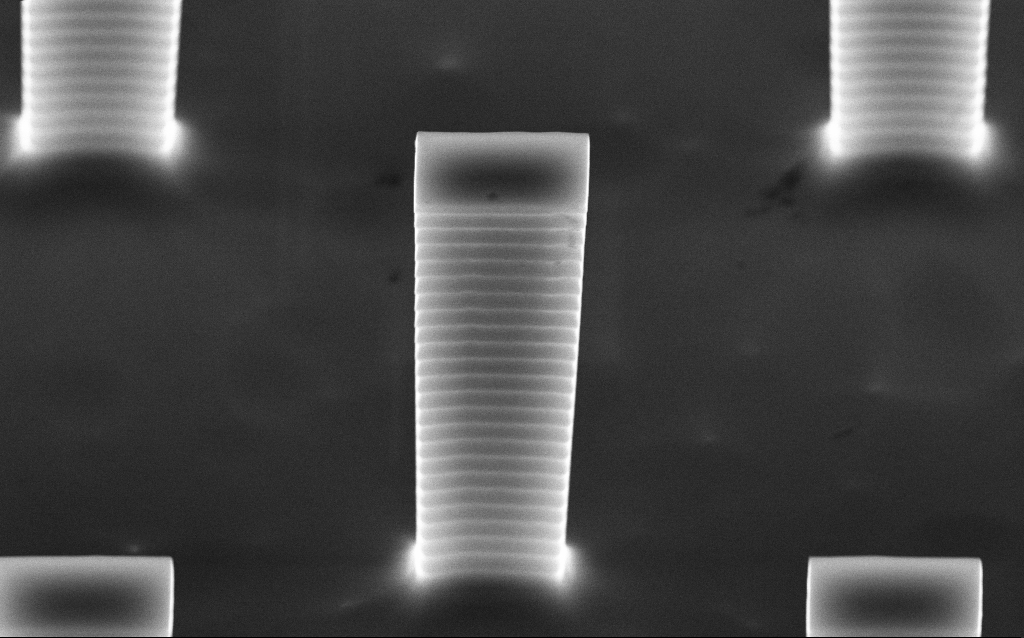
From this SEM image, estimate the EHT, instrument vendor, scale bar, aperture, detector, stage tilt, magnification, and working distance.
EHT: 10 kV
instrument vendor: Zeiss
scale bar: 2000 nm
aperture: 30 µm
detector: InLens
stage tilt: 45°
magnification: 24.65 K X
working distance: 5.1 mm